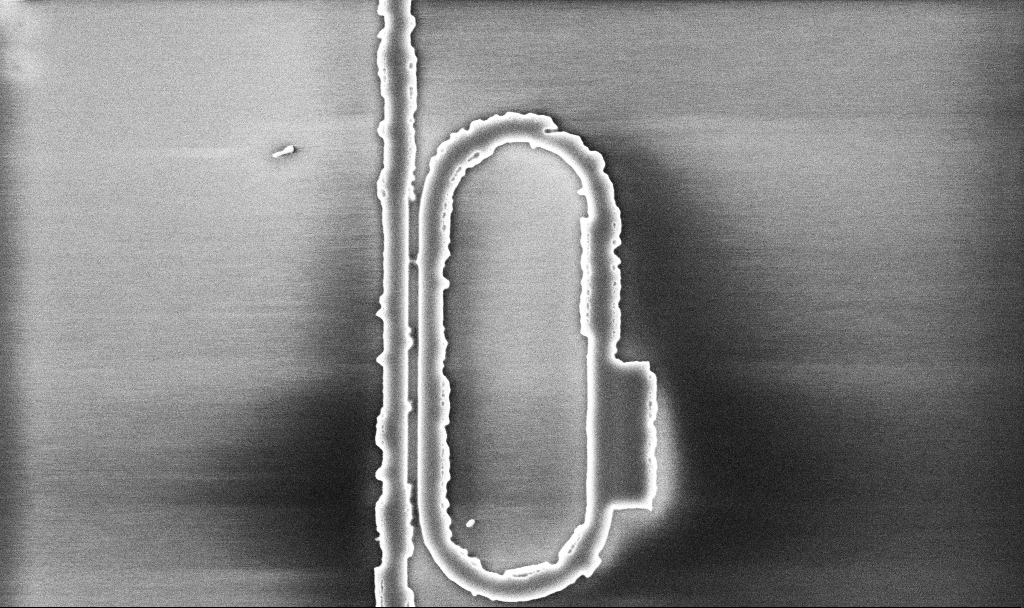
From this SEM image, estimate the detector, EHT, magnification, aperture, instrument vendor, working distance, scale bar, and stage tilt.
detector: InLens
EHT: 5 kV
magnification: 17.93 K X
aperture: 30 µm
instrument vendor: Zeiss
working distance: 10.1 mm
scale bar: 1000 nm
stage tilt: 0°